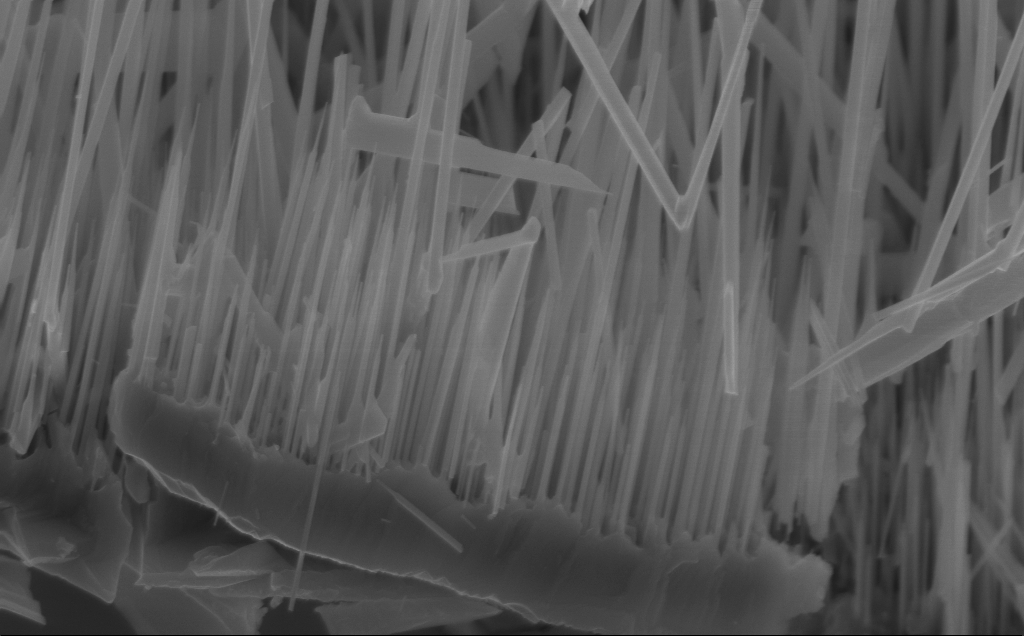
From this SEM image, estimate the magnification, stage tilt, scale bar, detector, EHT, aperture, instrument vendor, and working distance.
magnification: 37.19 K X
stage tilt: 45°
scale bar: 1000 nm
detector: InLens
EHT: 10 kV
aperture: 30 µm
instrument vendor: Zeiss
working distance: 5 mm